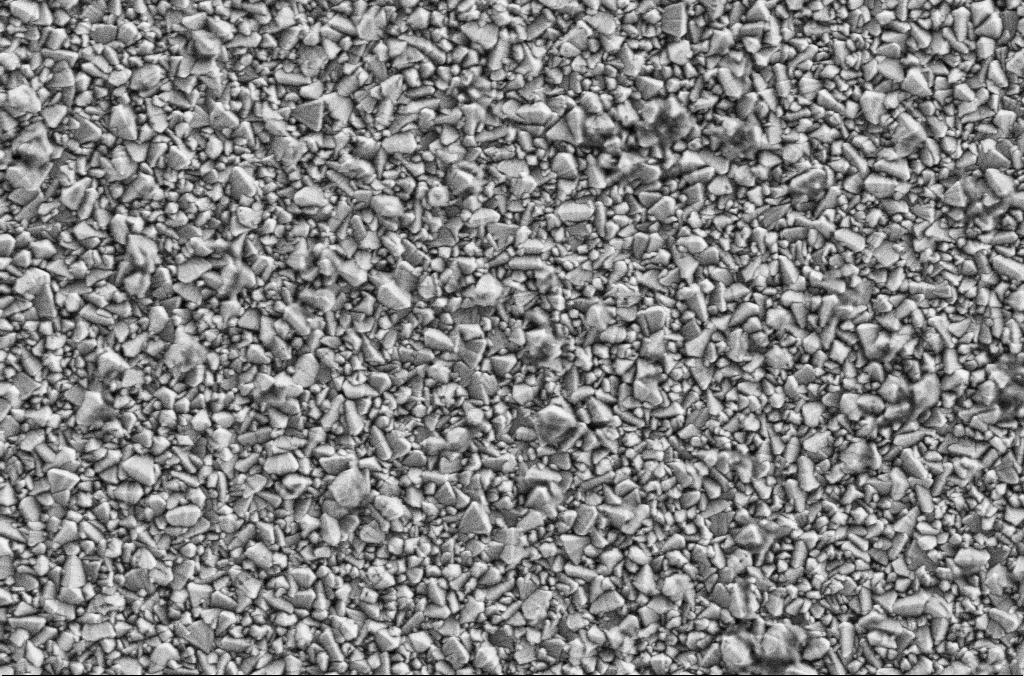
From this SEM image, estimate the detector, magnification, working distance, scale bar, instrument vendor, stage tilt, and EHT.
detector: SE2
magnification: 30 K X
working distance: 1.9 mm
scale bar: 2000 nm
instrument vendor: Zeiss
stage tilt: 0°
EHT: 2 kV